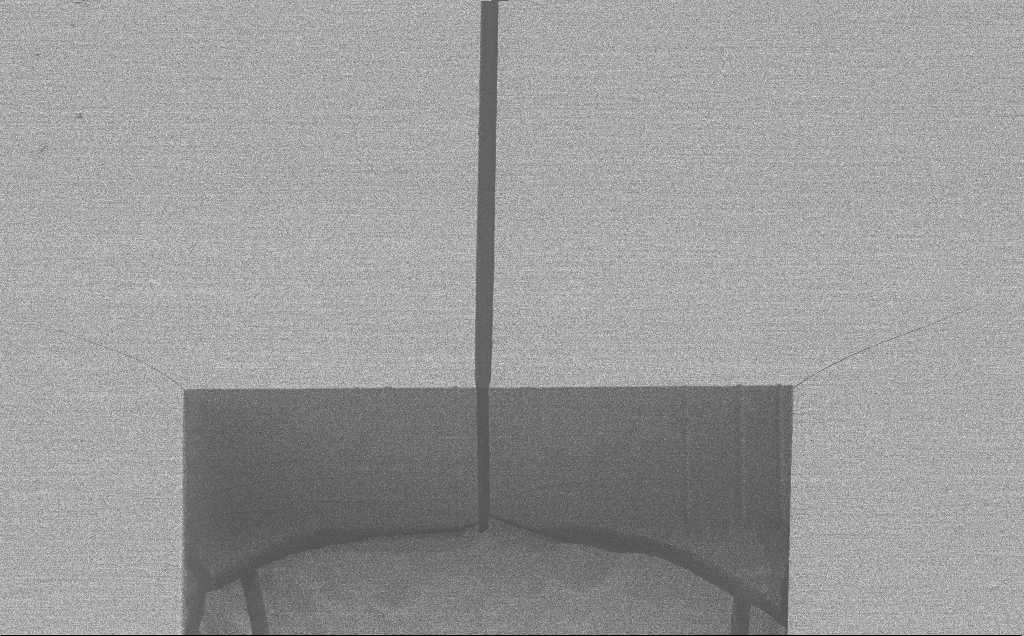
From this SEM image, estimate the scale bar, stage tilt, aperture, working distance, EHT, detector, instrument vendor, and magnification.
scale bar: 100000 nm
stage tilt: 45°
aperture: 30 µm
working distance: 6 mm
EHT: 1.2 kV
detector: SE2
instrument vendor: Zeiss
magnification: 0.56 K X